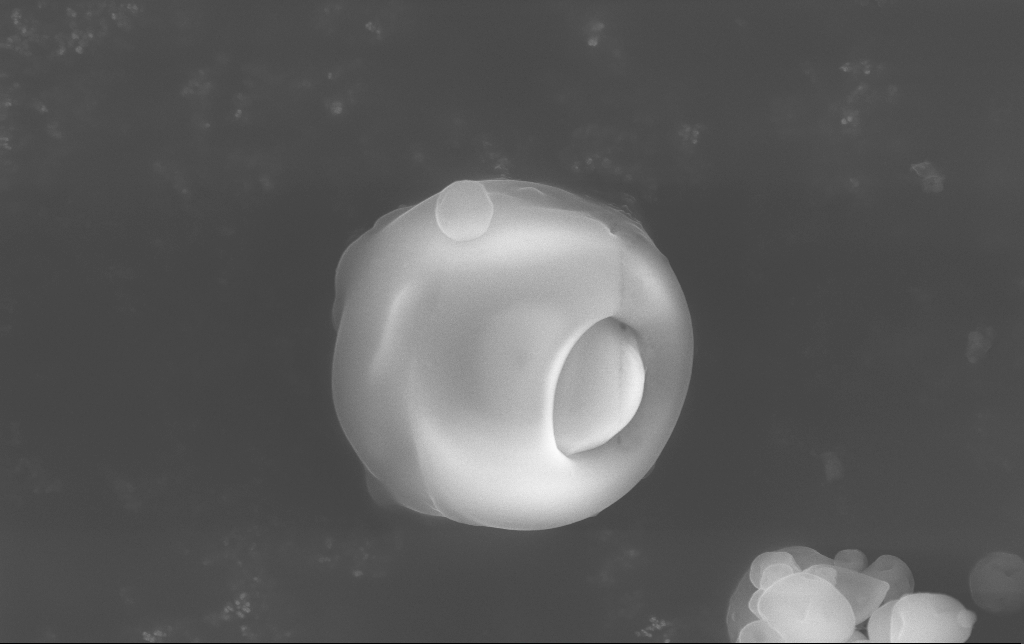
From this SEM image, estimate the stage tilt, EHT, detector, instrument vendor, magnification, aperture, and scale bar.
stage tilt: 0°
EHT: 15 kV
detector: InLens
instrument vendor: Zeiss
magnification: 25.35 K X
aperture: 30 µm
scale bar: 1000 nm